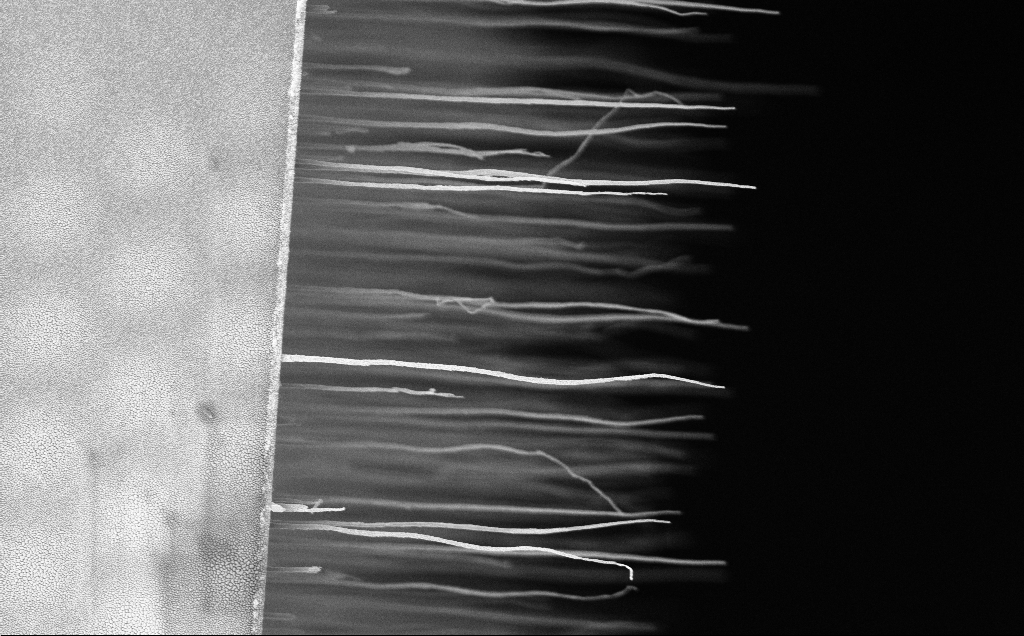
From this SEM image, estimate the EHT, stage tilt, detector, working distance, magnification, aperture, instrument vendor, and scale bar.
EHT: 5 kV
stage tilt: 0°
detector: InLens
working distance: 12 mm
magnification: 13.88 K X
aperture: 30 µm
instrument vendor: Zeiss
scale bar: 2000 nm